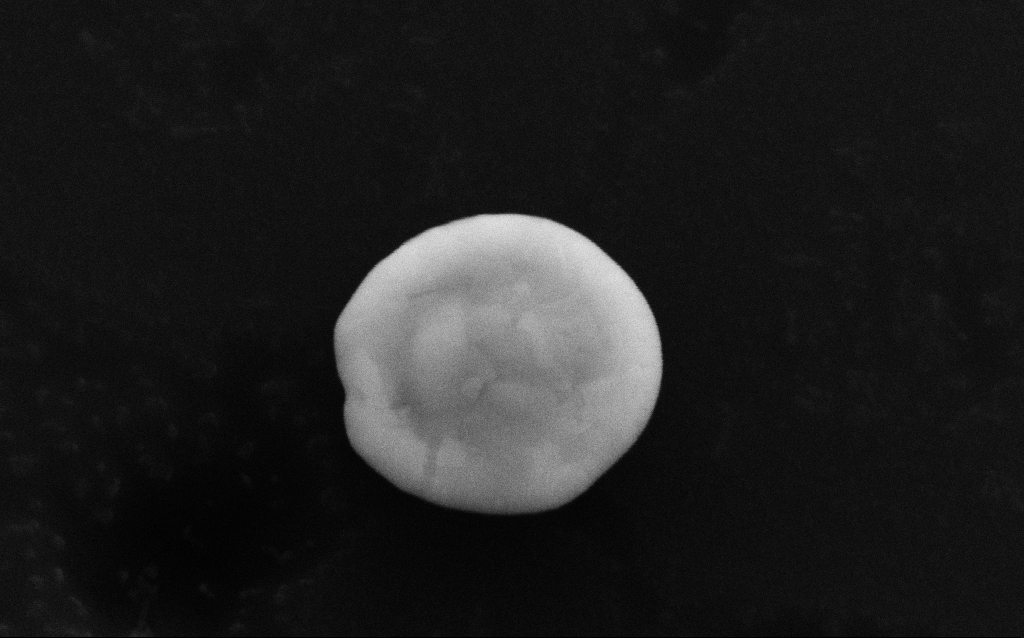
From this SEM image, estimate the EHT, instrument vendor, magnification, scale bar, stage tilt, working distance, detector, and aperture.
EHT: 10 kV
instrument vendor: Zeiss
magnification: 200.65 K X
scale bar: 200 nm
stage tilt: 21.3°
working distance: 6 mm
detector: SE2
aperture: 30 µm